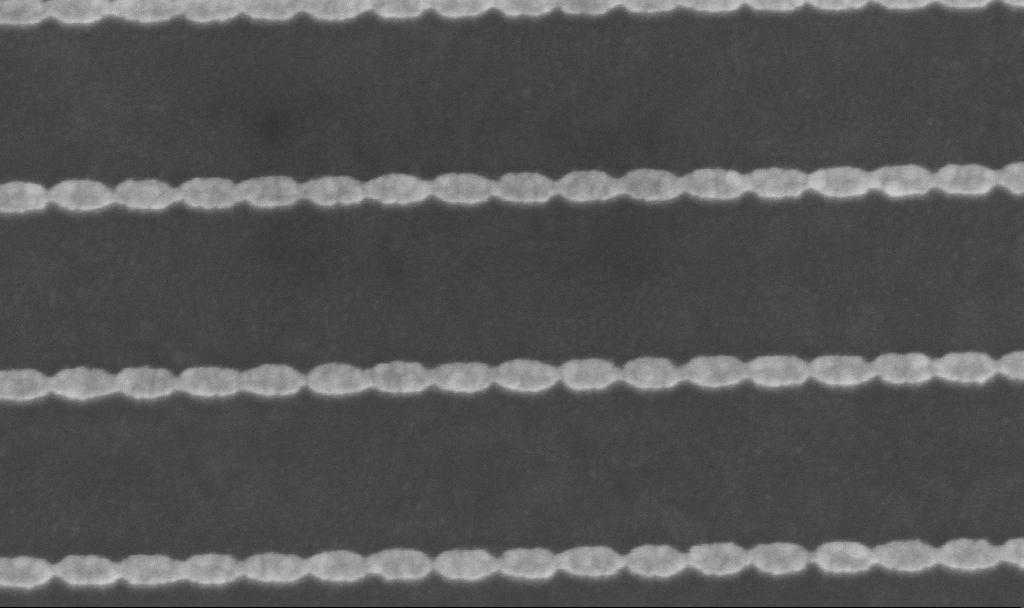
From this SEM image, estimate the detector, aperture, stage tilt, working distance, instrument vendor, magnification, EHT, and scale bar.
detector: InLens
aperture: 30 µm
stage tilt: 0°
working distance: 6 mm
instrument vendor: Zeiss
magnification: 231.7 K X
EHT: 5 kV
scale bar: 200 nm